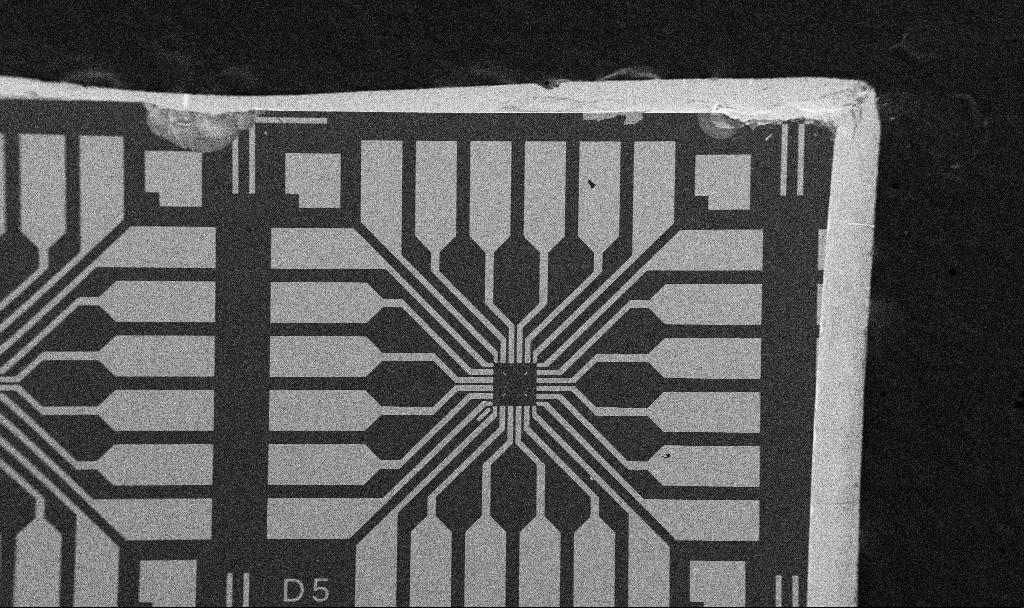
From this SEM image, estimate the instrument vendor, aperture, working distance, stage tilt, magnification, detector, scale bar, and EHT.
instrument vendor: Zeiss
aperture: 30 µm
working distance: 10.7 mm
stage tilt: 0°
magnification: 0.1 K X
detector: SE2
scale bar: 200000 nm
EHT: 5 kV